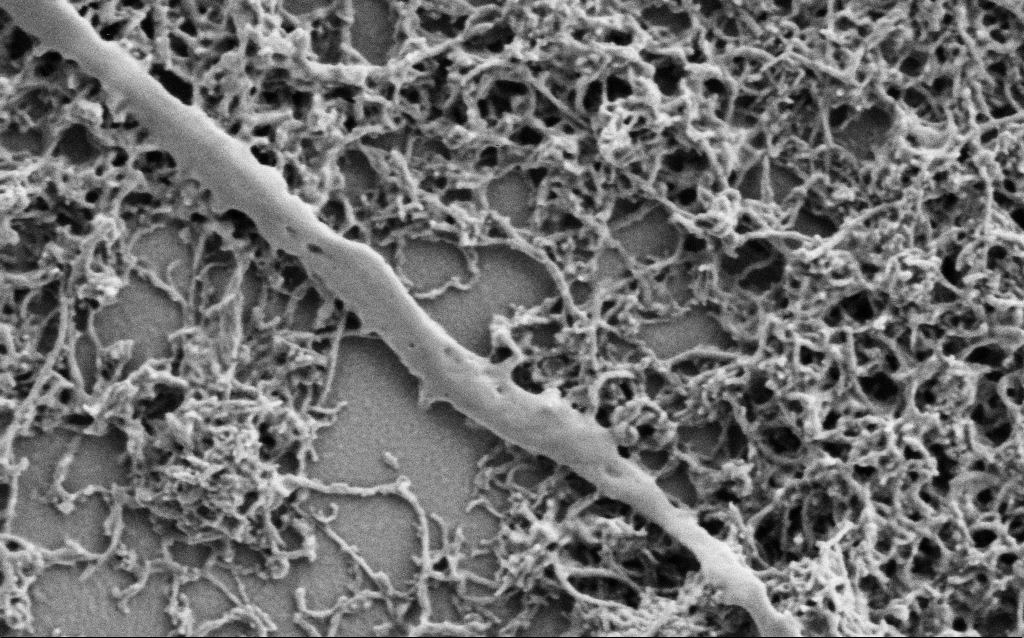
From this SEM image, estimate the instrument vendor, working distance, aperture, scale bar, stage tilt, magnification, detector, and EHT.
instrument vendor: Zeiss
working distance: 7 mm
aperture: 30 µm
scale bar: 200 nm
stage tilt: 0°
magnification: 75 K X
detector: SE2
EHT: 1 kV